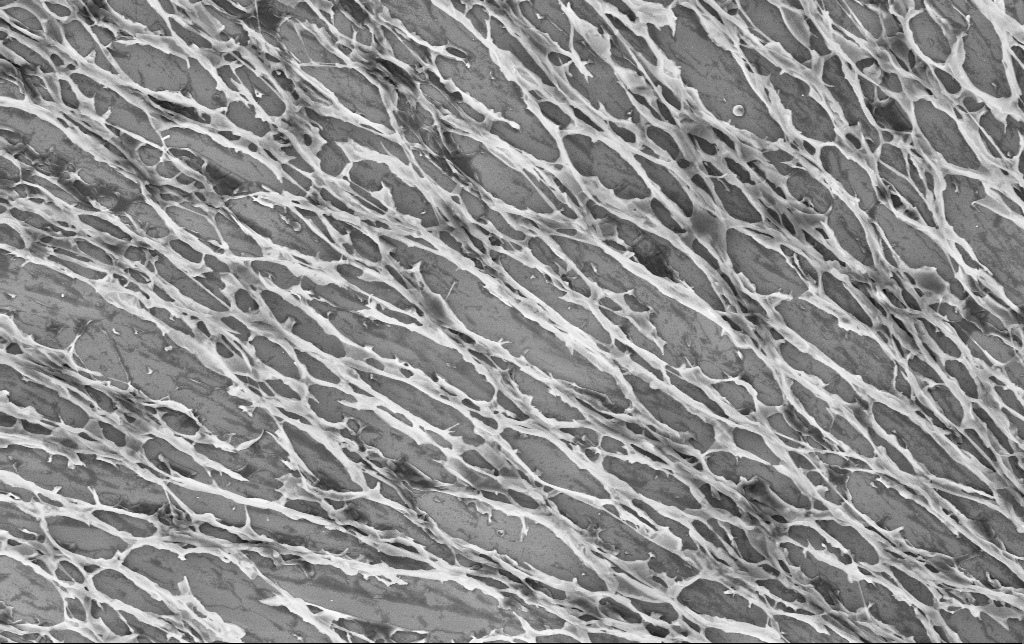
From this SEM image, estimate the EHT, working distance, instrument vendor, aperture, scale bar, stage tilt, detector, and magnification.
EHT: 3 kV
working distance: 3.4 mm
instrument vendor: Zeiss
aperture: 30 µm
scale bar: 2000 nm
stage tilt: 0°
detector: InLens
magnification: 10.54 K X